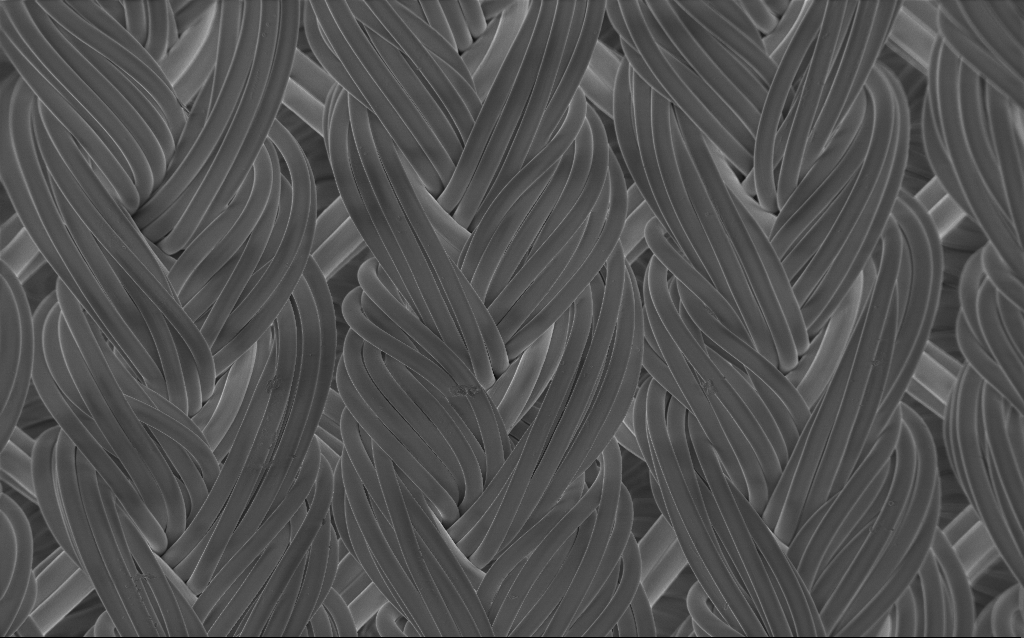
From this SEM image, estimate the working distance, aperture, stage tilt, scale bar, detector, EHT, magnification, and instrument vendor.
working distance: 4 mm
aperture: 30 µm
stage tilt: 0°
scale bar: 100000 nm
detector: InLens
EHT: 1 kV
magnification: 0.282 K X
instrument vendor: Zeiss